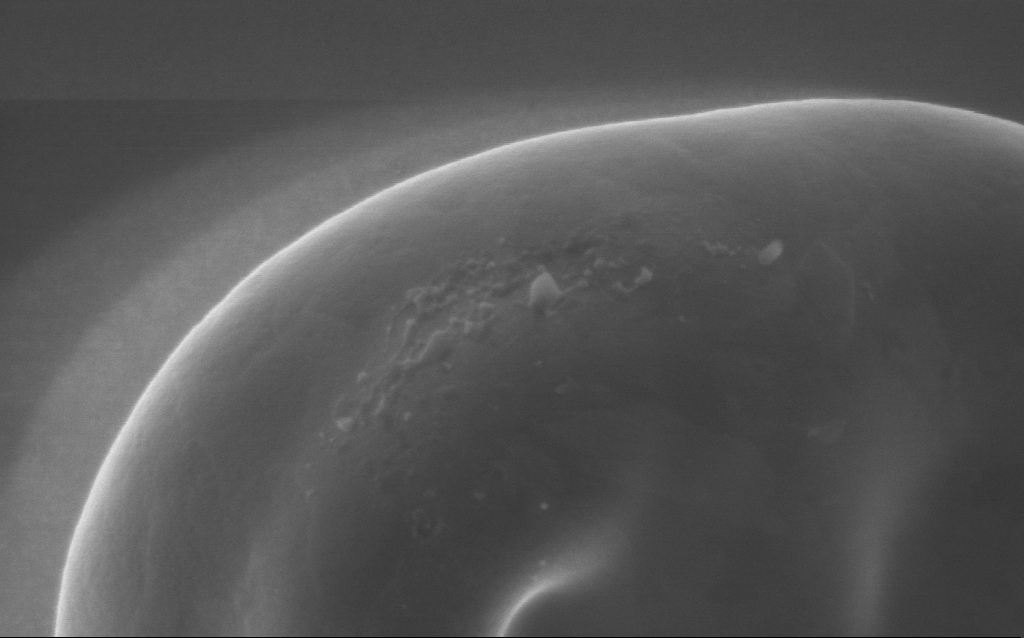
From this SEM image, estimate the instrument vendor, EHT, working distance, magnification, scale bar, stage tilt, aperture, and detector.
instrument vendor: Zeiss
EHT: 5 kV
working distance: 3 mm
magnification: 100 K X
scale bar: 200 nm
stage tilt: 0°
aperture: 30 µm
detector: InLens